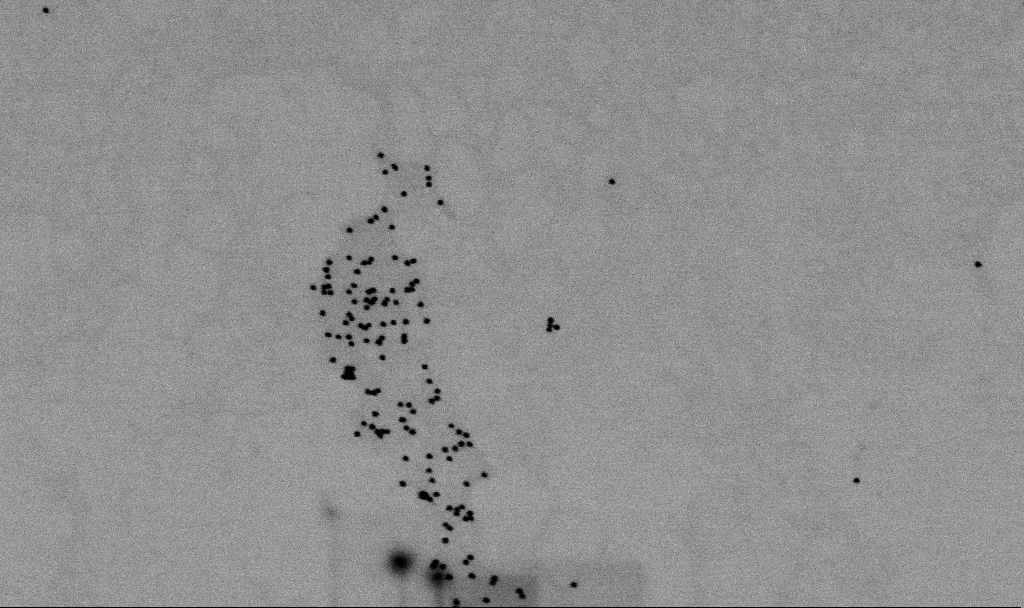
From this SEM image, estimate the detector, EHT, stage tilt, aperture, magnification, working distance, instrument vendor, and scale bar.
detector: SE2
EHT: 2 kV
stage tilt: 0°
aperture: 30 µm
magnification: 80 K X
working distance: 5.5 mm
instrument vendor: Zeiss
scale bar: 200 nm